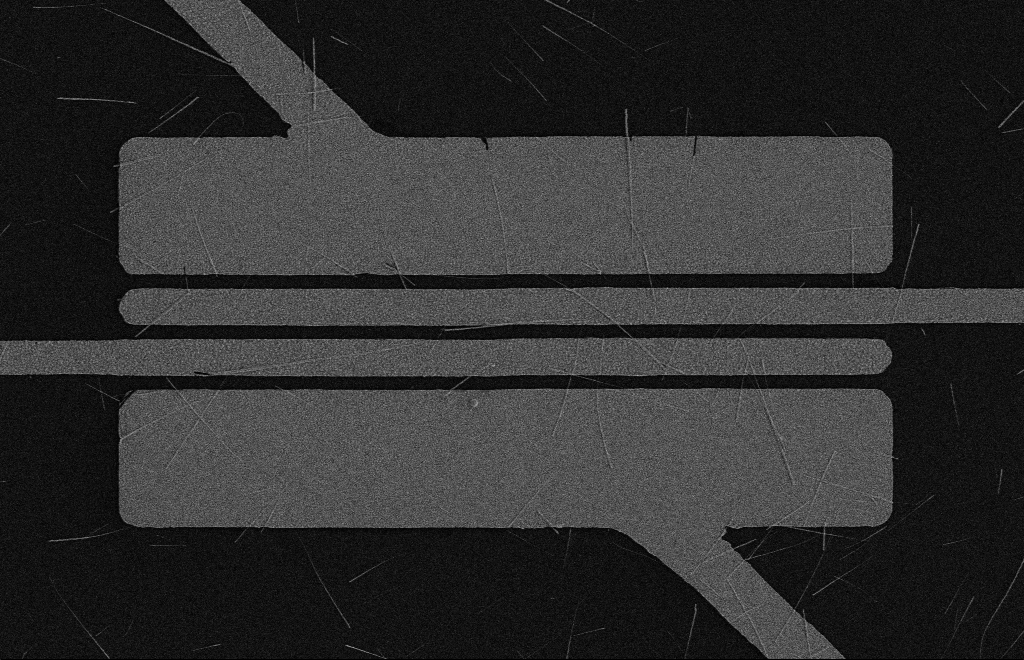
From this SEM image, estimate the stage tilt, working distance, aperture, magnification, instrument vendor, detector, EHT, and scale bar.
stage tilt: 0°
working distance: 16 mm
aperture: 10 µm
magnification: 4.66 K X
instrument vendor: Zeiss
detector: SE2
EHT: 5 kV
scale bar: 10000 nm